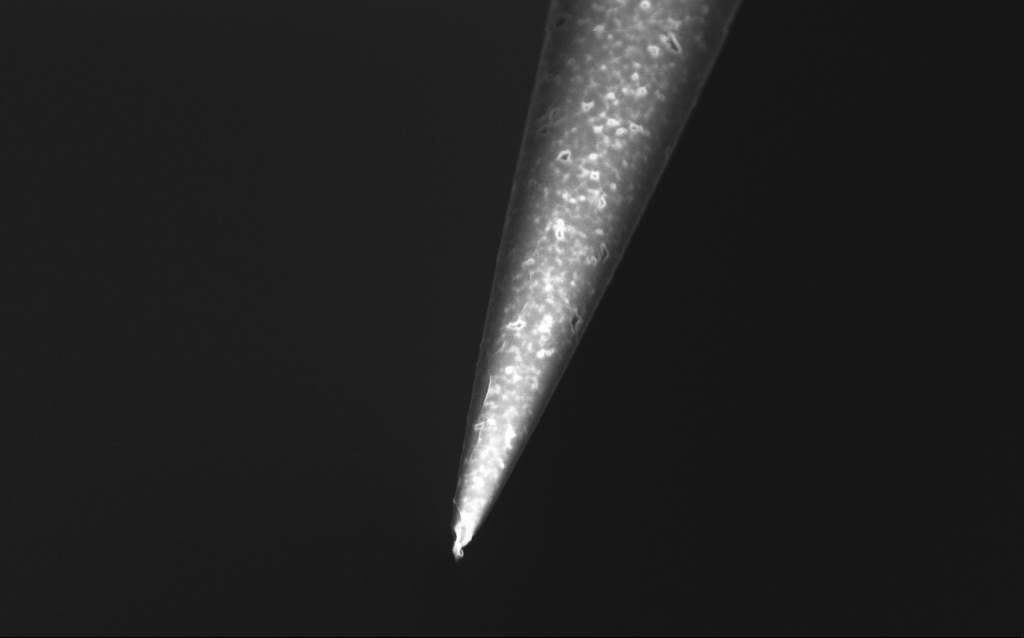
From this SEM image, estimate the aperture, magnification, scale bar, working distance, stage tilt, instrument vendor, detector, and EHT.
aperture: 30 µm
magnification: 25 K X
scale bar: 2000 nm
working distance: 6 mm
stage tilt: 45°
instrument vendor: Zeiss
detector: InLens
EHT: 1 kV